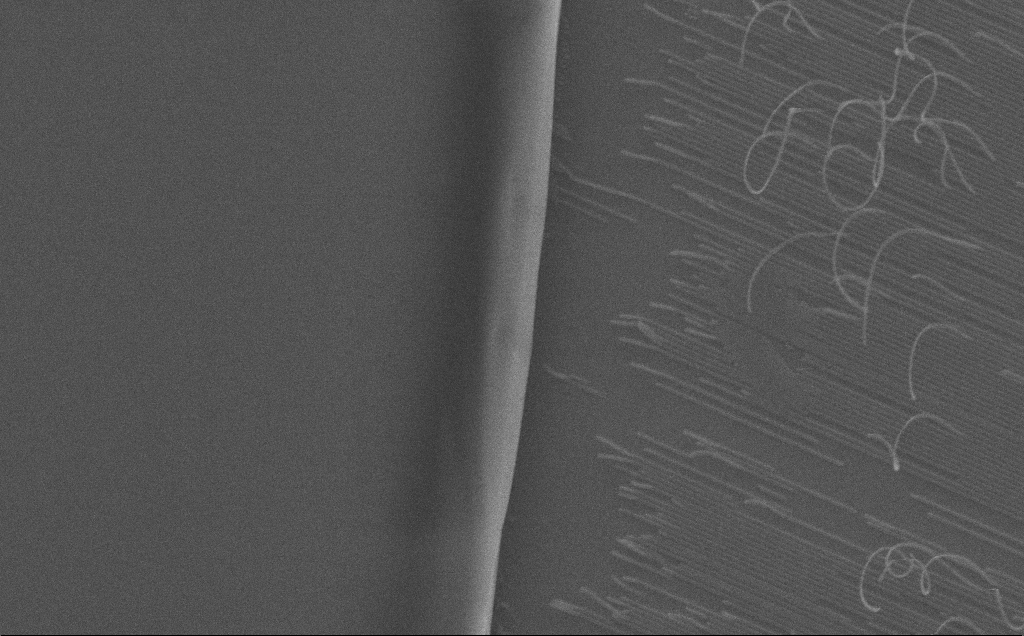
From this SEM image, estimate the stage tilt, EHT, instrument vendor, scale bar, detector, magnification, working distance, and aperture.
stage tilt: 0°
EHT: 10 kV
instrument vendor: Zeiss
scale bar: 2000 nm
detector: SE2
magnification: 8.03 K X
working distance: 12 mm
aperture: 30 µm